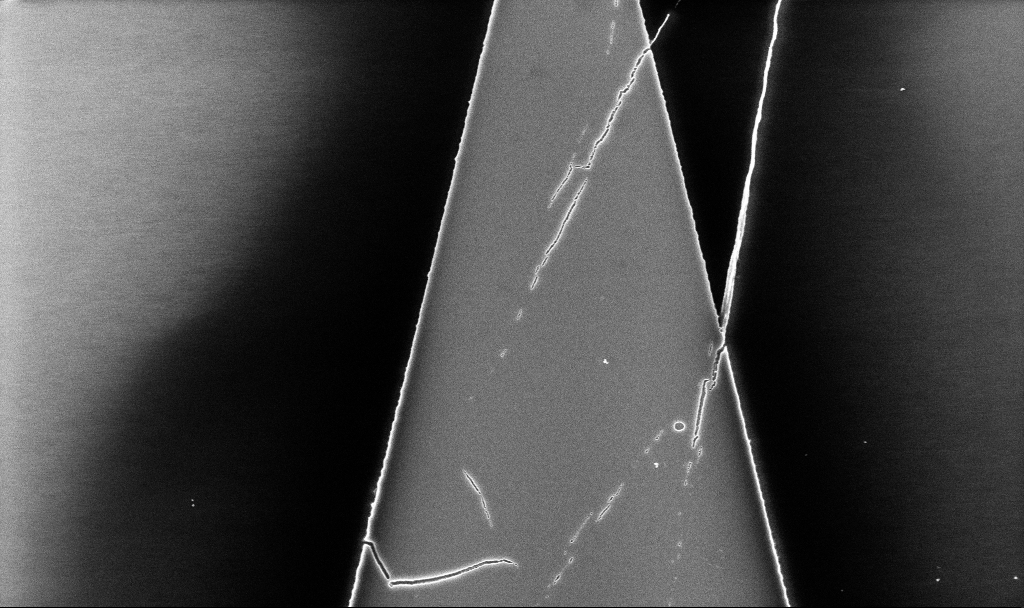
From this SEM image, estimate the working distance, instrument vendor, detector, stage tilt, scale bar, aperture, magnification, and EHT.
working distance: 5.2 mm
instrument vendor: Zeiss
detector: InLens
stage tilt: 0°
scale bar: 2000 nm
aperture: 30 µm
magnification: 10.37 K X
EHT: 5 kV